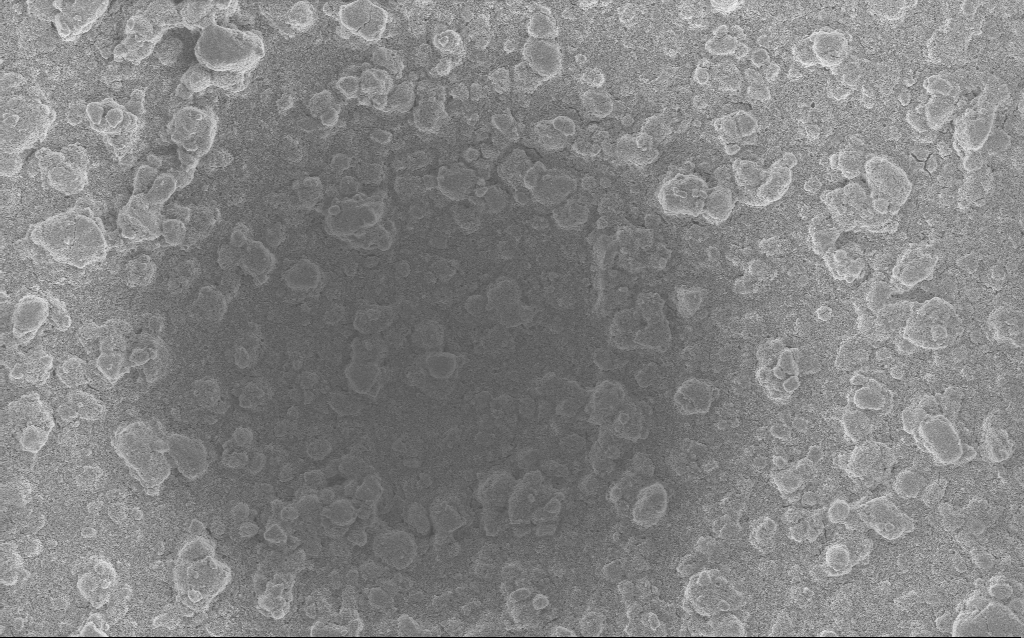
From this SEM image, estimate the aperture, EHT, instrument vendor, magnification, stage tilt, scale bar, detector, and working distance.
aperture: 20 µm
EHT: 5 kV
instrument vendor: Zeiss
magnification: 1.69 K X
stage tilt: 0°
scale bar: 10000 nm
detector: InLens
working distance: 2.6 mm